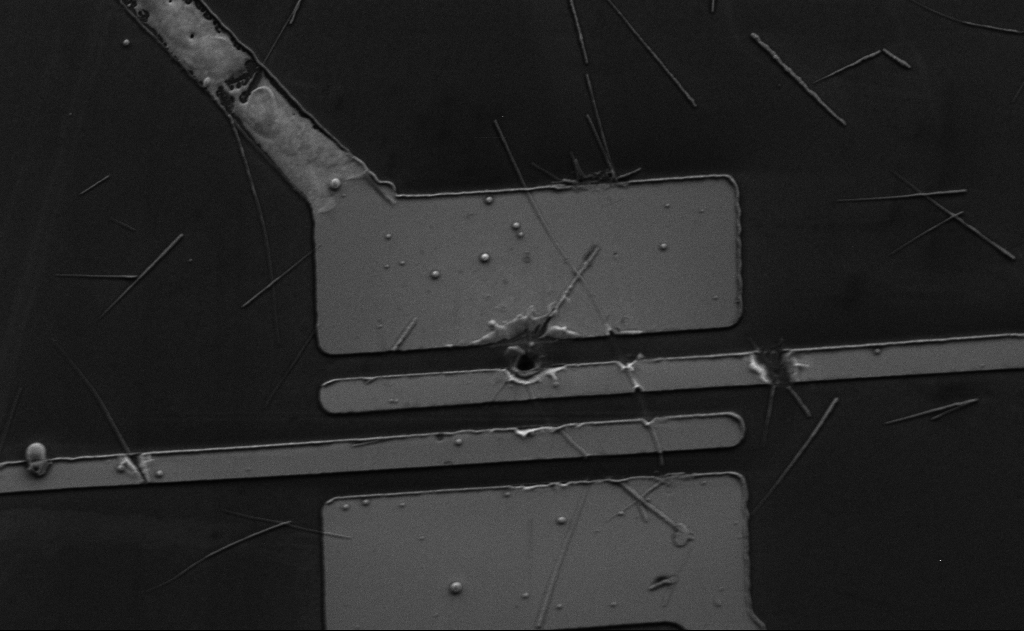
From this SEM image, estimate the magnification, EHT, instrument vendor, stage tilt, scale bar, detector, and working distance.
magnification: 5.31 K X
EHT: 5 kV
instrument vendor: Zeiss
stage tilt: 0°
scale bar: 10000 nm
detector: SE2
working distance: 15 mm